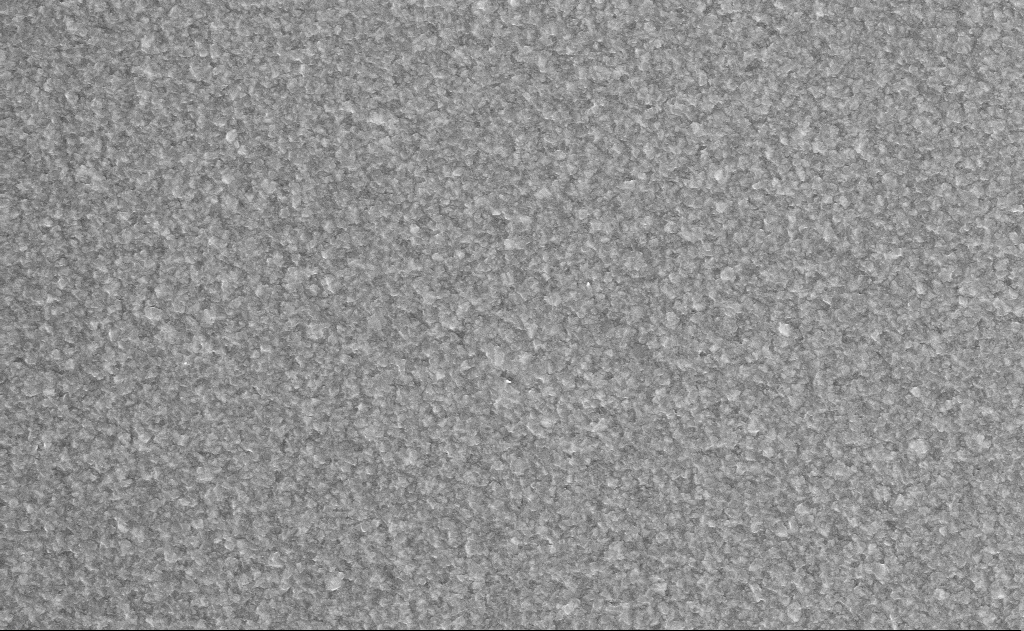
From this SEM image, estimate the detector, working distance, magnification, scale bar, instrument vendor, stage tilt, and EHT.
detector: InLens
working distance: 16 mm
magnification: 20 K X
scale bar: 1000 nm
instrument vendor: Zeiss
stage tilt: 0°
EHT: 10 kV